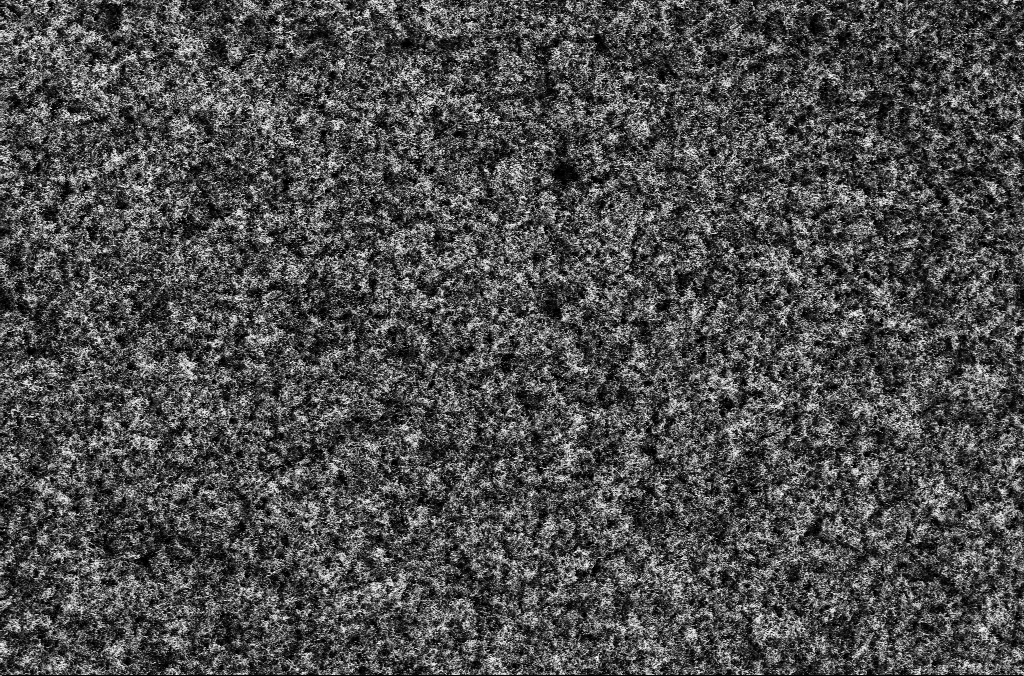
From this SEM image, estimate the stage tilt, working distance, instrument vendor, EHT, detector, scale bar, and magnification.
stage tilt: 0°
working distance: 5.3 mm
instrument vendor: Zeiss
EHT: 1.8 kV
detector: SE2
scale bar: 10000 nm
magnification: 2.5 K X